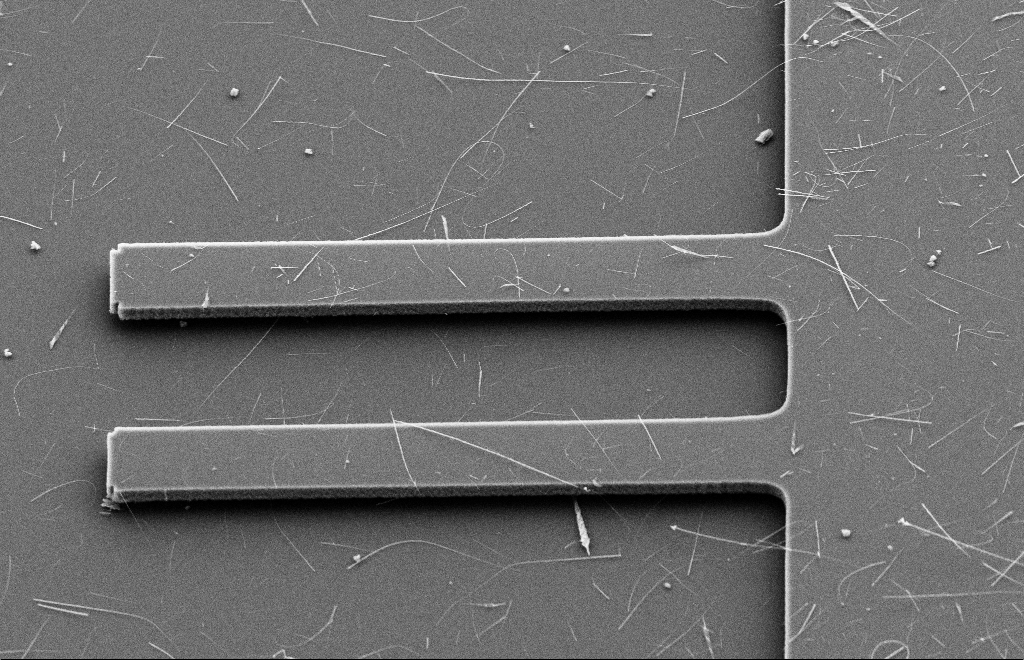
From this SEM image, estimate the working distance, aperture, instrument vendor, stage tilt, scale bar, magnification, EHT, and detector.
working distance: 9 mm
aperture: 20 µm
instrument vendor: Zeiss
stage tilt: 39.8°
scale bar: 10000 nm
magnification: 2.5 K X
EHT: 10 kV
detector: SE2